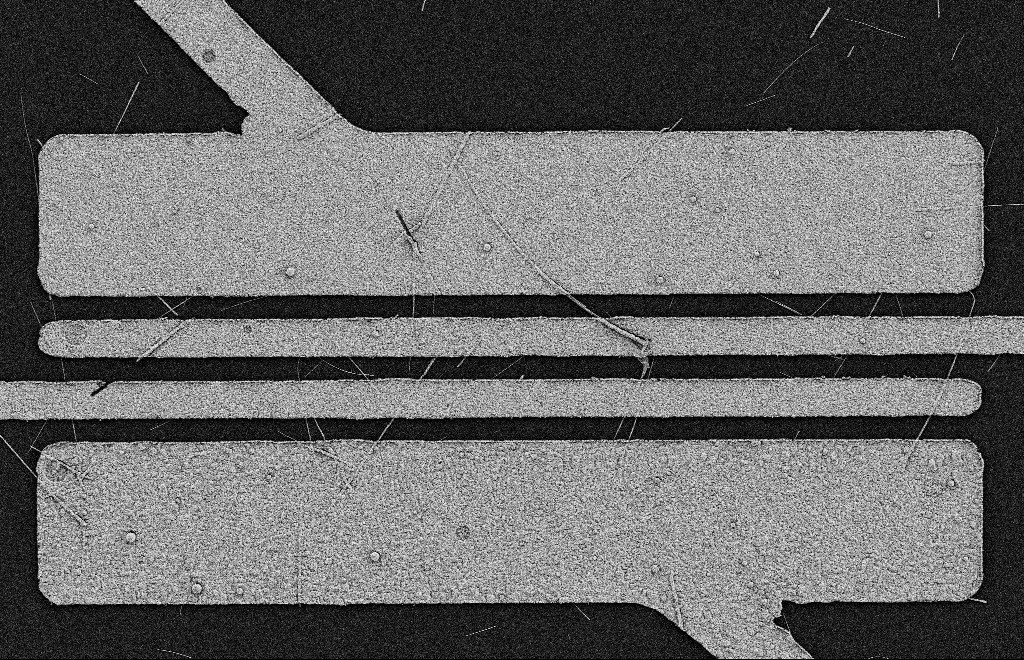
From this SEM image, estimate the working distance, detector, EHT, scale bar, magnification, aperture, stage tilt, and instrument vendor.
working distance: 11 mm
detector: SE2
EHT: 2 kV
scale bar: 2000 nm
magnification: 5.64 K X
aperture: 20 µm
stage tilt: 0°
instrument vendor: Zeiss